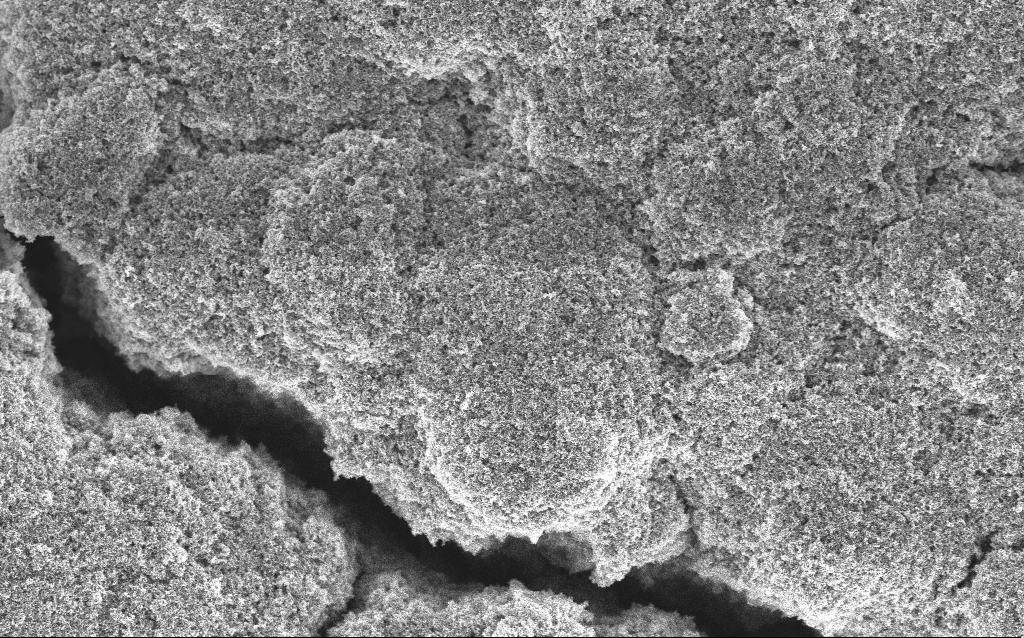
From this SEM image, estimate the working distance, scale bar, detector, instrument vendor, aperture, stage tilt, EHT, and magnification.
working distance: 4.2 mm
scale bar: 1000 nm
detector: InLens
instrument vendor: Zeiss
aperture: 30 µm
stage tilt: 0°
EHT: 5 kV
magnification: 15.33 K X